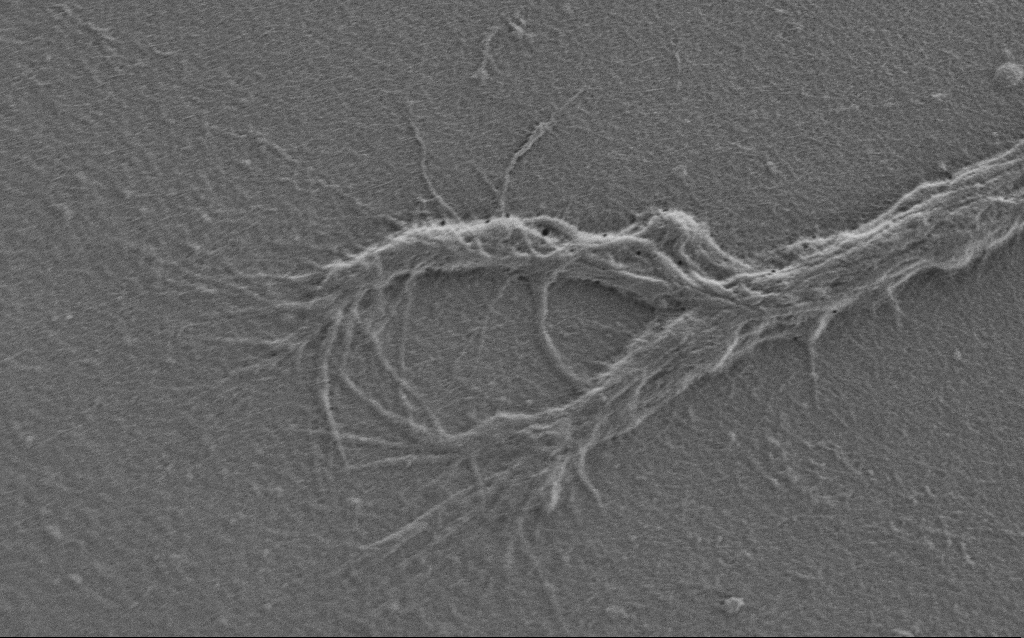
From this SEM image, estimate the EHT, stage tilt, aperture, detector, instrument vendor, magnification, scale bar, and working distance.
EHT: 0.9 kV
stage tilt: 0°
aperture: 30 µm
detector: SE2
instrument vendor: Zeiss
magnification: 7.5 K X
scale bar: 2000 nm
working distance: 6 mm